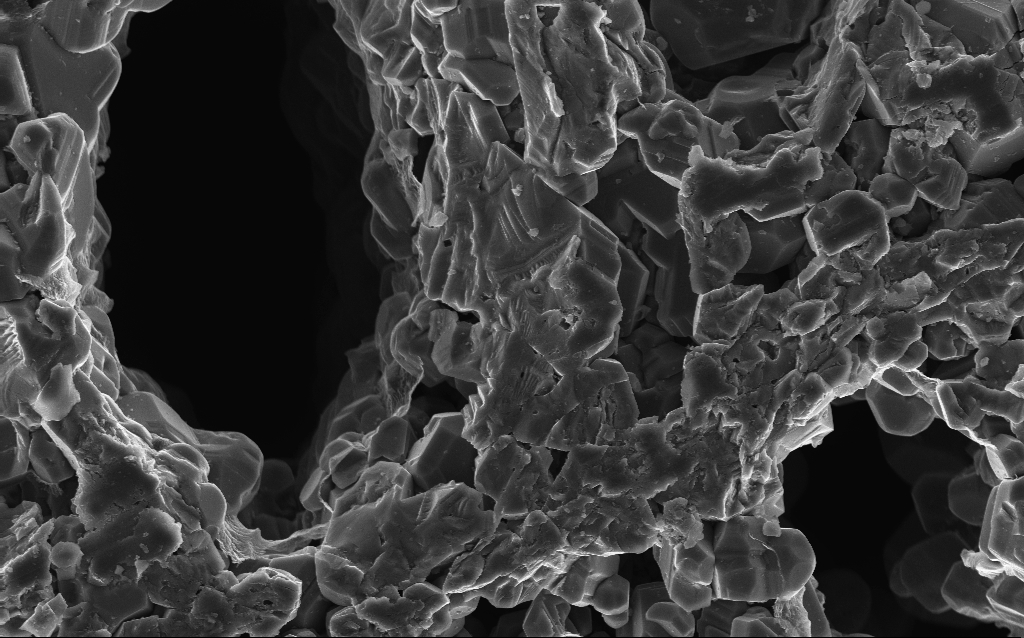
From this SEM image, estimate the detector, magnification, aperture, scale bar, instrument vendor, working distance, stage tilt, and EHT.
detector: InLens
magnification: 5 K X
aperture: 30 µm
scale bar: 10000 nm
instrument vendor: Zeiss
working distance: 3 mm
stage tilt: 0°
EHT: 10 kV